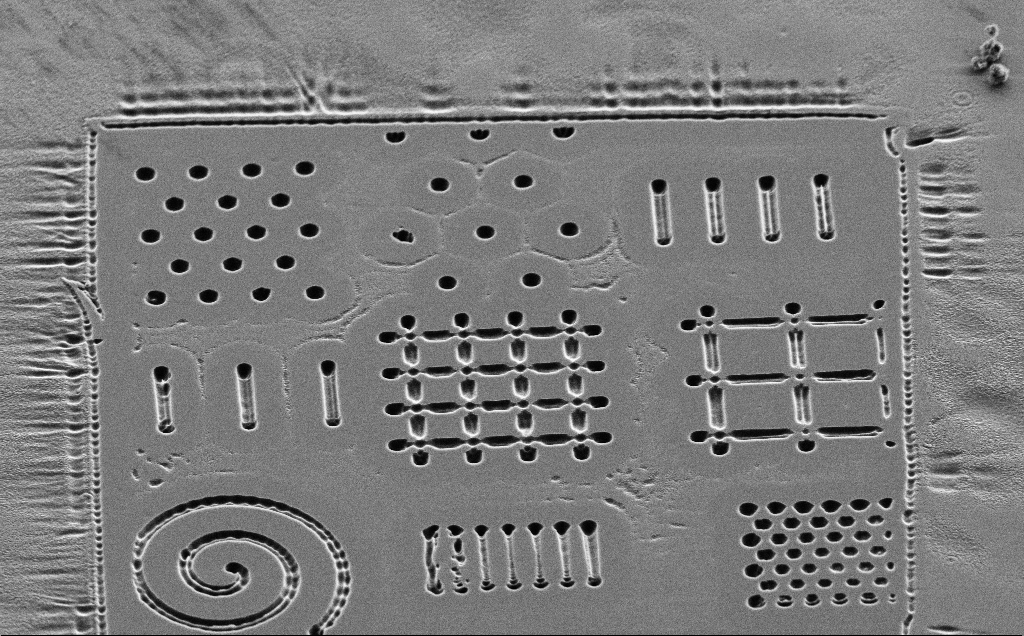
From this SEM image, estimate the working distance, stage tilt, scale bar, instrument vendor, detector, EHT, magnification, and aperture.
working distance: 10 mm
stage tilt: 45°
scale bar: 10000 nm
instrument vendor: Zeiss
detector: SE2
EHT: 5 kV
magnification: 3.07 K X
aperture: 30 µm